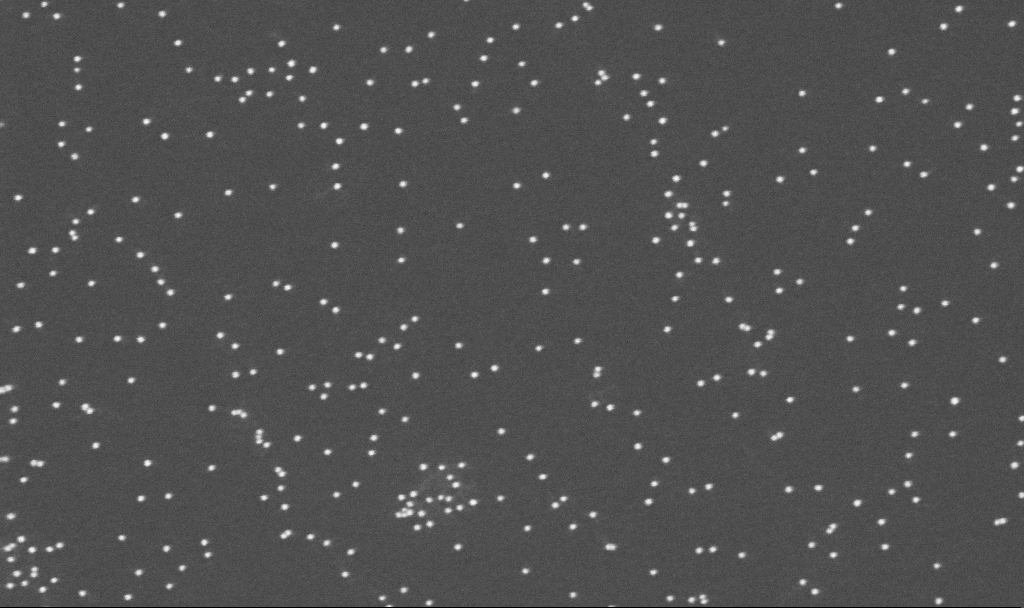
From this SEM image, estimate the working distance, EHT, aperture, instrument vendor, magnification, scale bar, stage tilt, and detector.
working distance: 3.3 mm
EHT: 10 kV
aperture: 30 µm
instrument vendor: Zeiss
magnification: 200 K X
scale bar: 200 nm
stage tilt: -0°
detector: InLens